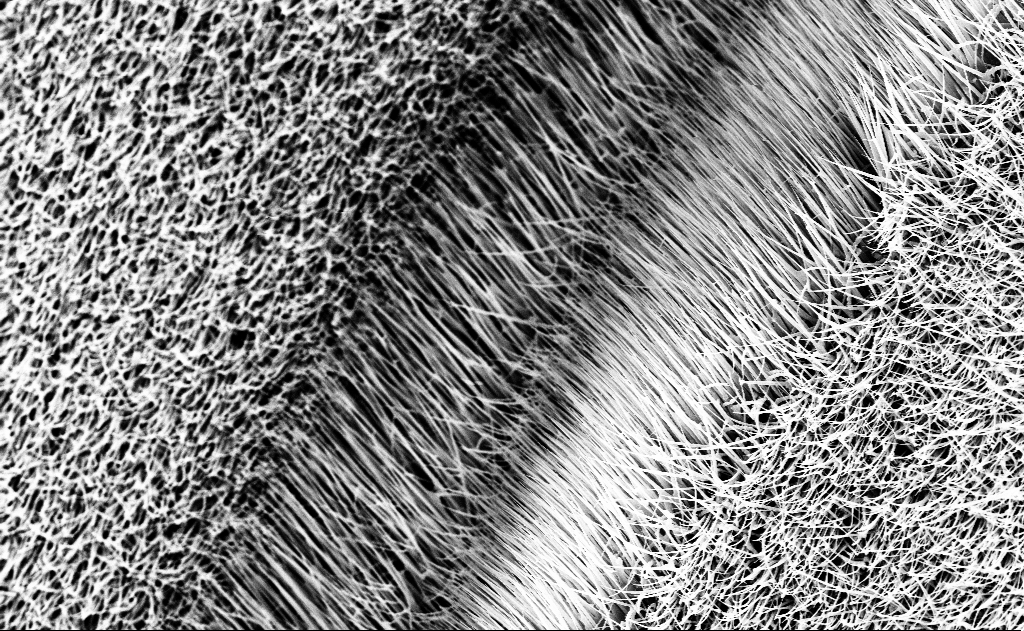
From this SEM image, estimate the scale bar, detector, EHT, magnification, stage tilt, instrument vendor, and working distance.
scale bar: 2000 nm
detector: InLens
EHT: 10 kV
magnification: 10 K X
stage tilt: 0°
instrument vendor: Zeiss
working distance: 12 mm